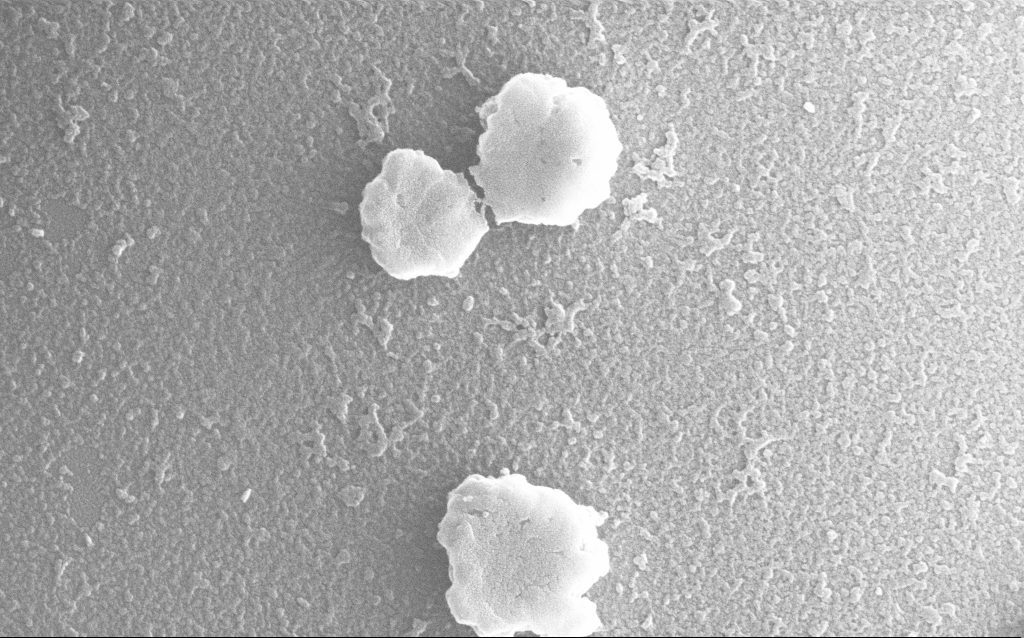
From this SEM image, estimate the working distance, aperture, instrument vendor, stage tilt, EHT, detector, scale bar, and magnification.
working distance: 1.6 mm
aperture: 30 µm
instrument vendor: Zeiss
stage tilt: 0°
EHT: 20 kV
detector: InLens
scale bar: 200 nm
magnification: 100 K X